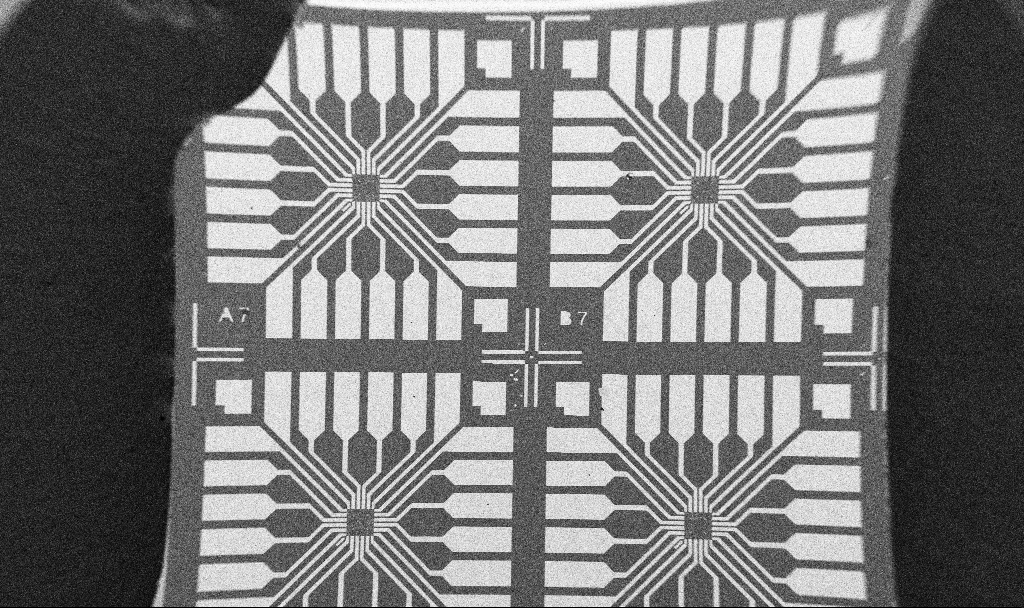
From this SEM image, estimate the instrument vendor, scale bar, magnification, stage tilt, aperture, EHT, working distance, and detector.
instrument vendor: Zeiss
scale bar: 1e+06 nm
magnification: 0.061 K X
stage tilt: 0°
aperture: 30 µm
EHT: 5 kV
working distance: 10.7 mm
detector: SE2